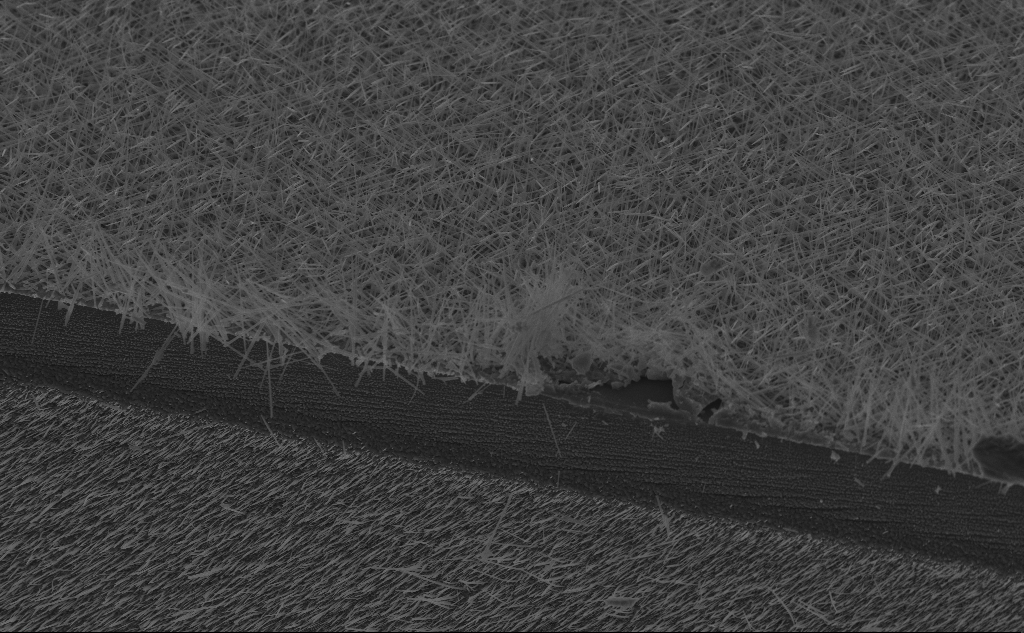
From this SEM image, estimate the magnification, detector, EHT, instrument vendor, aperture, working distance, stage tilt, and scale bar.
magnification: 6.82 K X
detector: InLens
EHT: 10 kV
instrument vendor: Zeiss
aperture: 30 µm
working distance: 5 mm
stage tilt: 45°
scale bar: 10000 nm